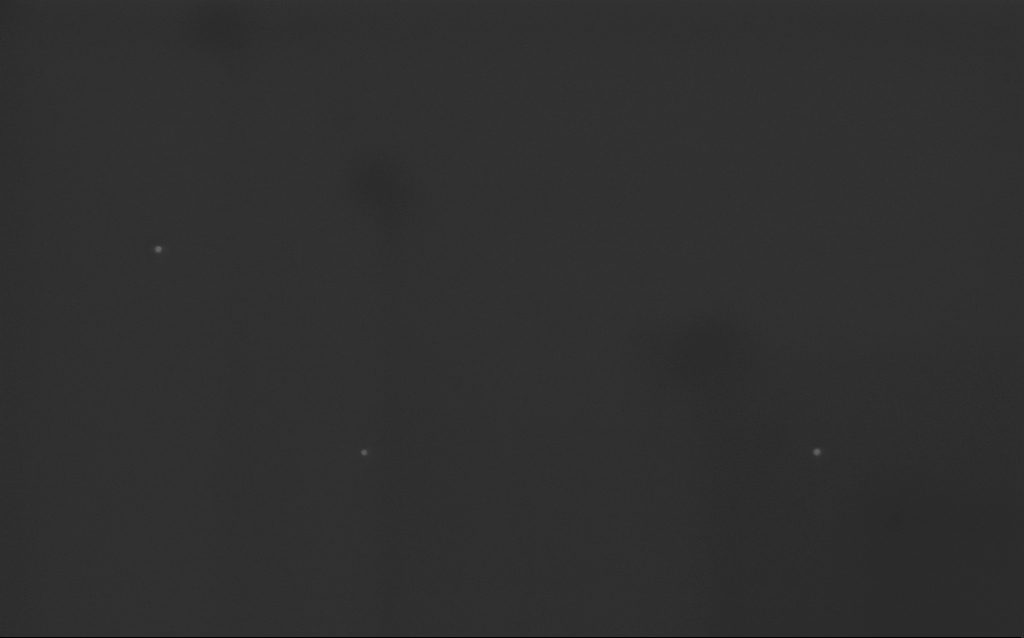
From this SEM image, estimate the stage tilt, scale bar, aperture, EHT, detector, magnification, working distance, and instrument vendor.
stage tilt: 0°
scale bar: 200 nm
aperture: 30 µm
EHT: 3 kV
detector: InLens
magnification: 86.12 K X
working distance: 3 mm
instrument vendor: Zeiss